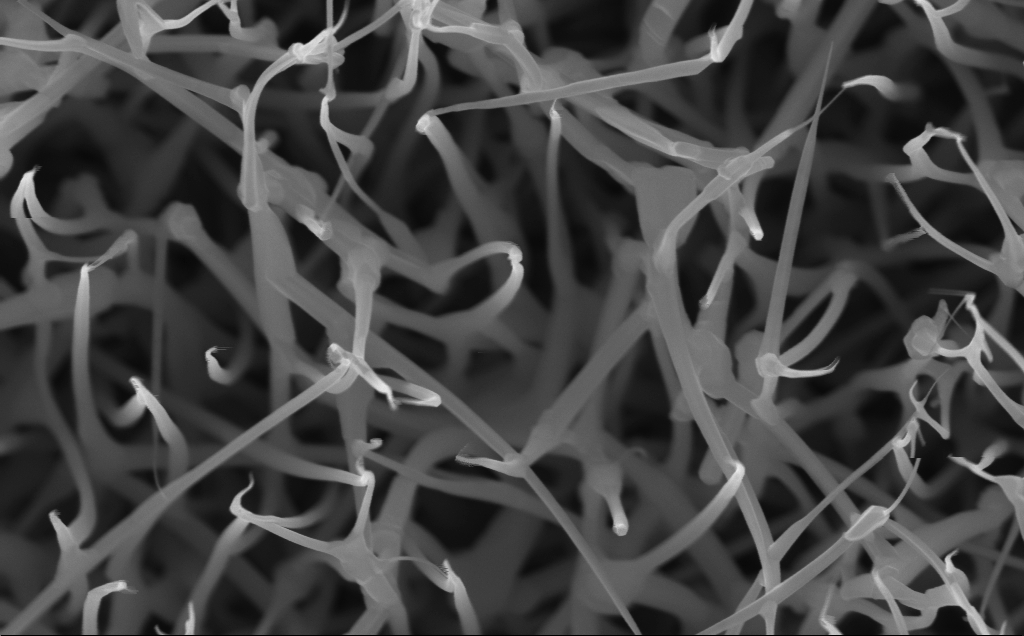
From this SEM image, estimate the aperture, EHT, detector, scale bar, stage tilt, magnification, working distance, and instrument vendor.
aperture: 30 µm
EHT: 10 kV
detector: InLens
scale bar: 200 nm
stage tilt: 0°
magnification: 80 K X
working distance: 5 mm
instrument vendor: Zeiss